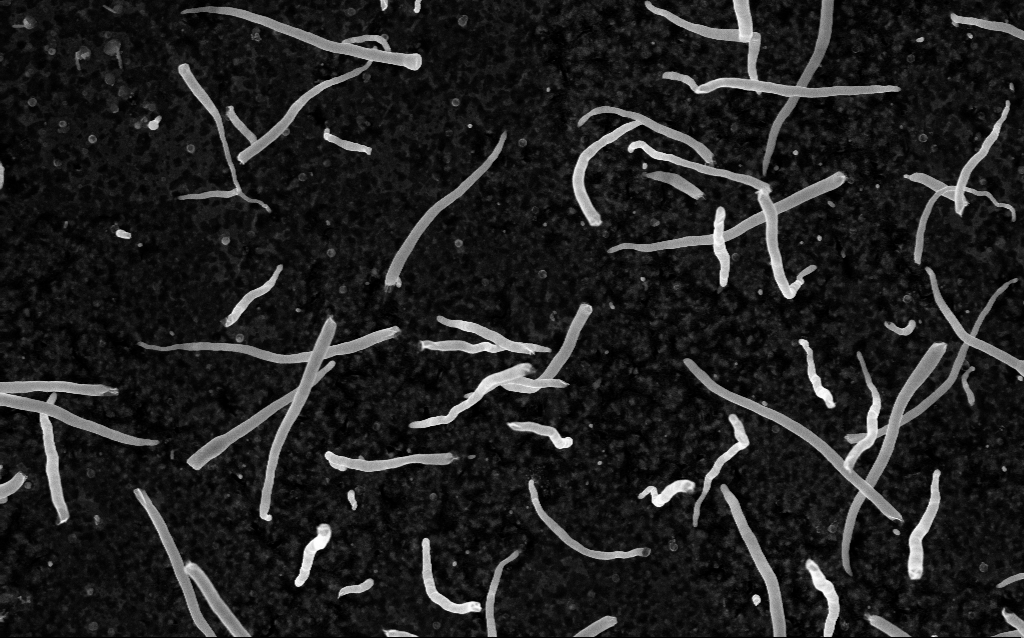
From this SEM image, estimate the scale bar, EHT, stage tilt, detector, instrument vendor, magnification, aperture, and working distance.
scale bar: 1000 nm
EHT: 5 kV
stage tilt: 0°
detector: InLens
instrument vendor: Zeiss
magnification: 50 K X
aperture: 30 µm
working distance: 2 mm